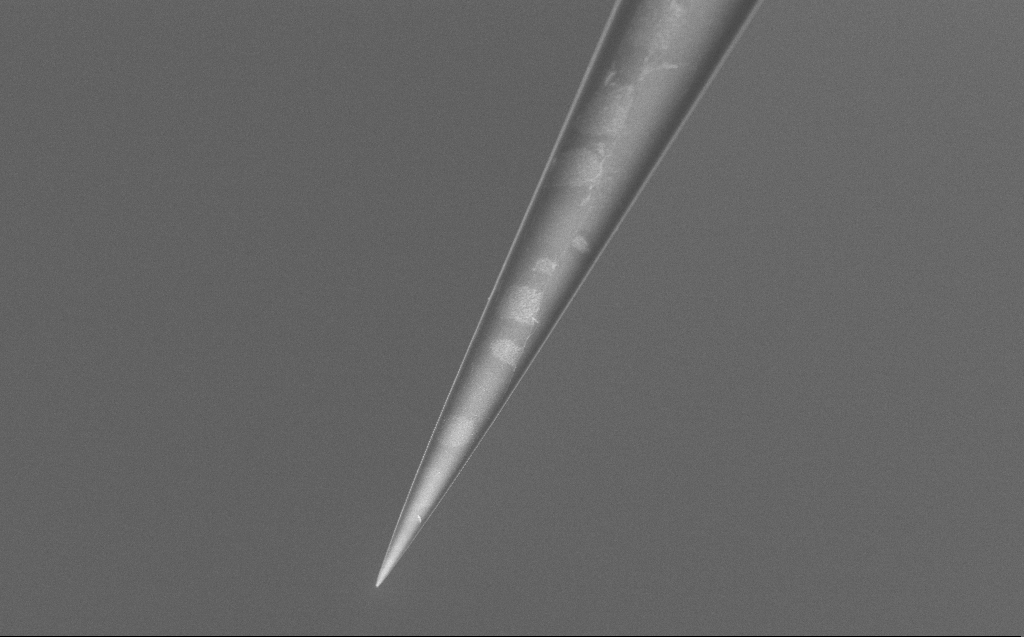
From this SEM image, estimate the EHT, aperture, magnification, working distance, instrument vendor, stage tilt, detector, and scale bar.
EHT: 5 kV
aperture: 30 µm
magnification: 10 K X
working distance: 6 mm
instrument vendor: Zeiss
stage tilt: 45°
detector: InLens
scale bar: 2000 nm